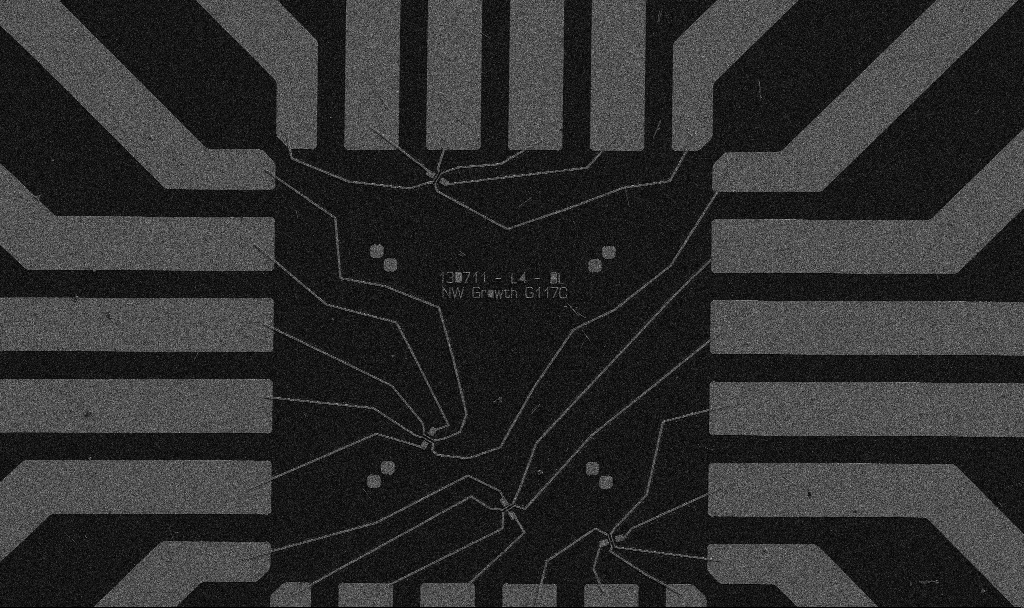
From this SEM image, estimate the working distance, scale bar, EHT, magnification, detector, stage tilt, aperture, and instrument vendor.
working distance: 10.7 mm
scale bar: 20000 nm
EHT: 5 kV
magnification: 1 K X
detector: SE2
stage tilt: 0°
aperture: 30 µm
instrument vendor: Zeiss